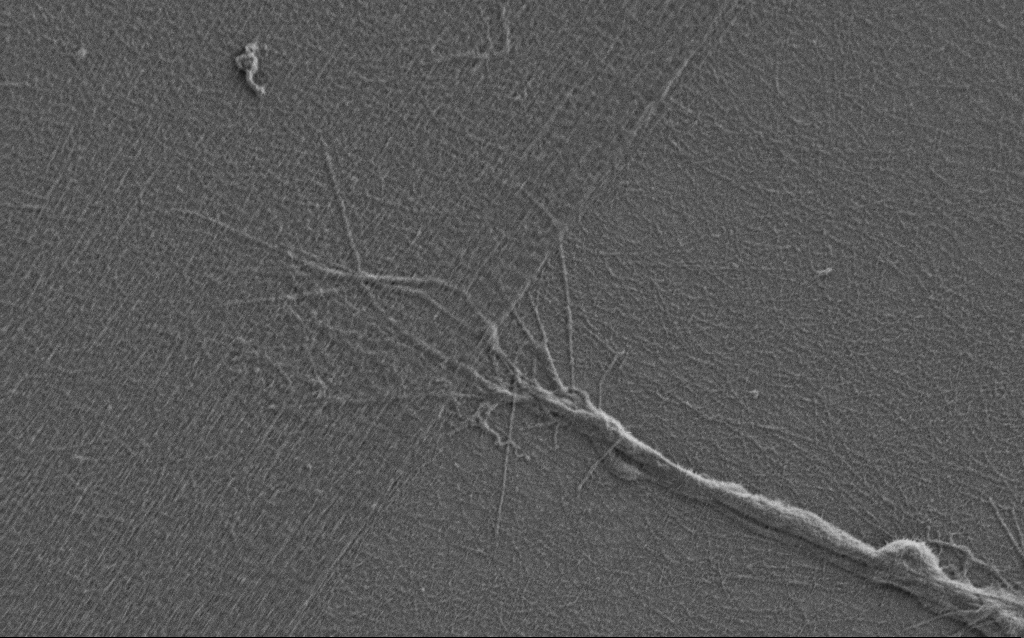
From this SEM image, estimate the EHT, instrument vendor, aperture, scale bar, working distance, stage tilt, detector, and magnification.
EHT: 0.9 kV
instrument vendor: Zeiss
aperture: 30 µm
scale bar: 2000 nm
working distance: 7 mm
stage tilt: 0°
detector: SE2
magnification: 10 K X